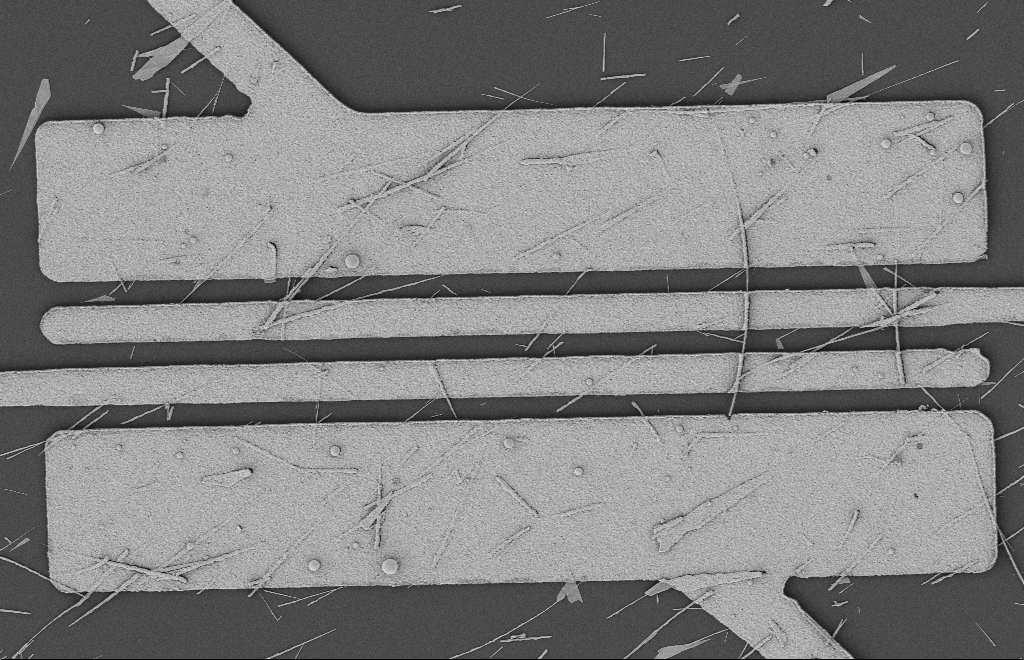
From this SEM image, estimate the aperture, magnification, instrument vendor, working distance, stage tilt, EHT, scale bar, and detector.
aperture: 20 µm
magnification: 5.67 K X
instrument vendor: Zeiss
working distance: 12 mm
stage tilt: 0°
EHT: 2 kV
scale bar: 2000 nm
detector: SE2